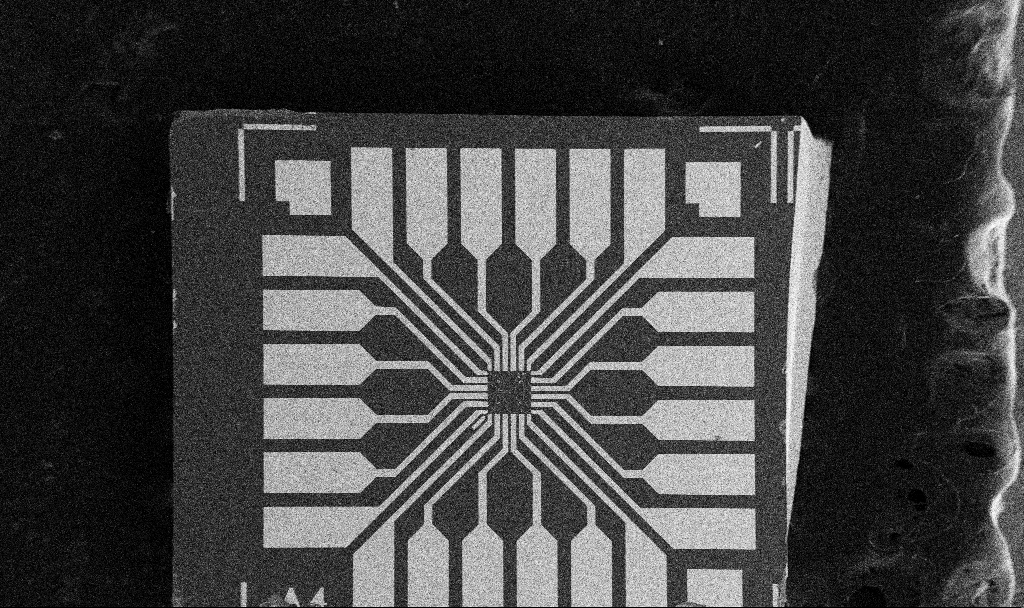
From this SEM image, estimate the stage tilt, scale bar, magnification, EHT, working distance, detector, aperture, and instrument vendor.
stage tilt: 0°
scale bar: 200000 nm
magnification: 0.1 K X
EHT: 5 kV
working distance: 10.6 mm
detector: SE2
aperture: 30 µm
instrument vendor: Zeiss